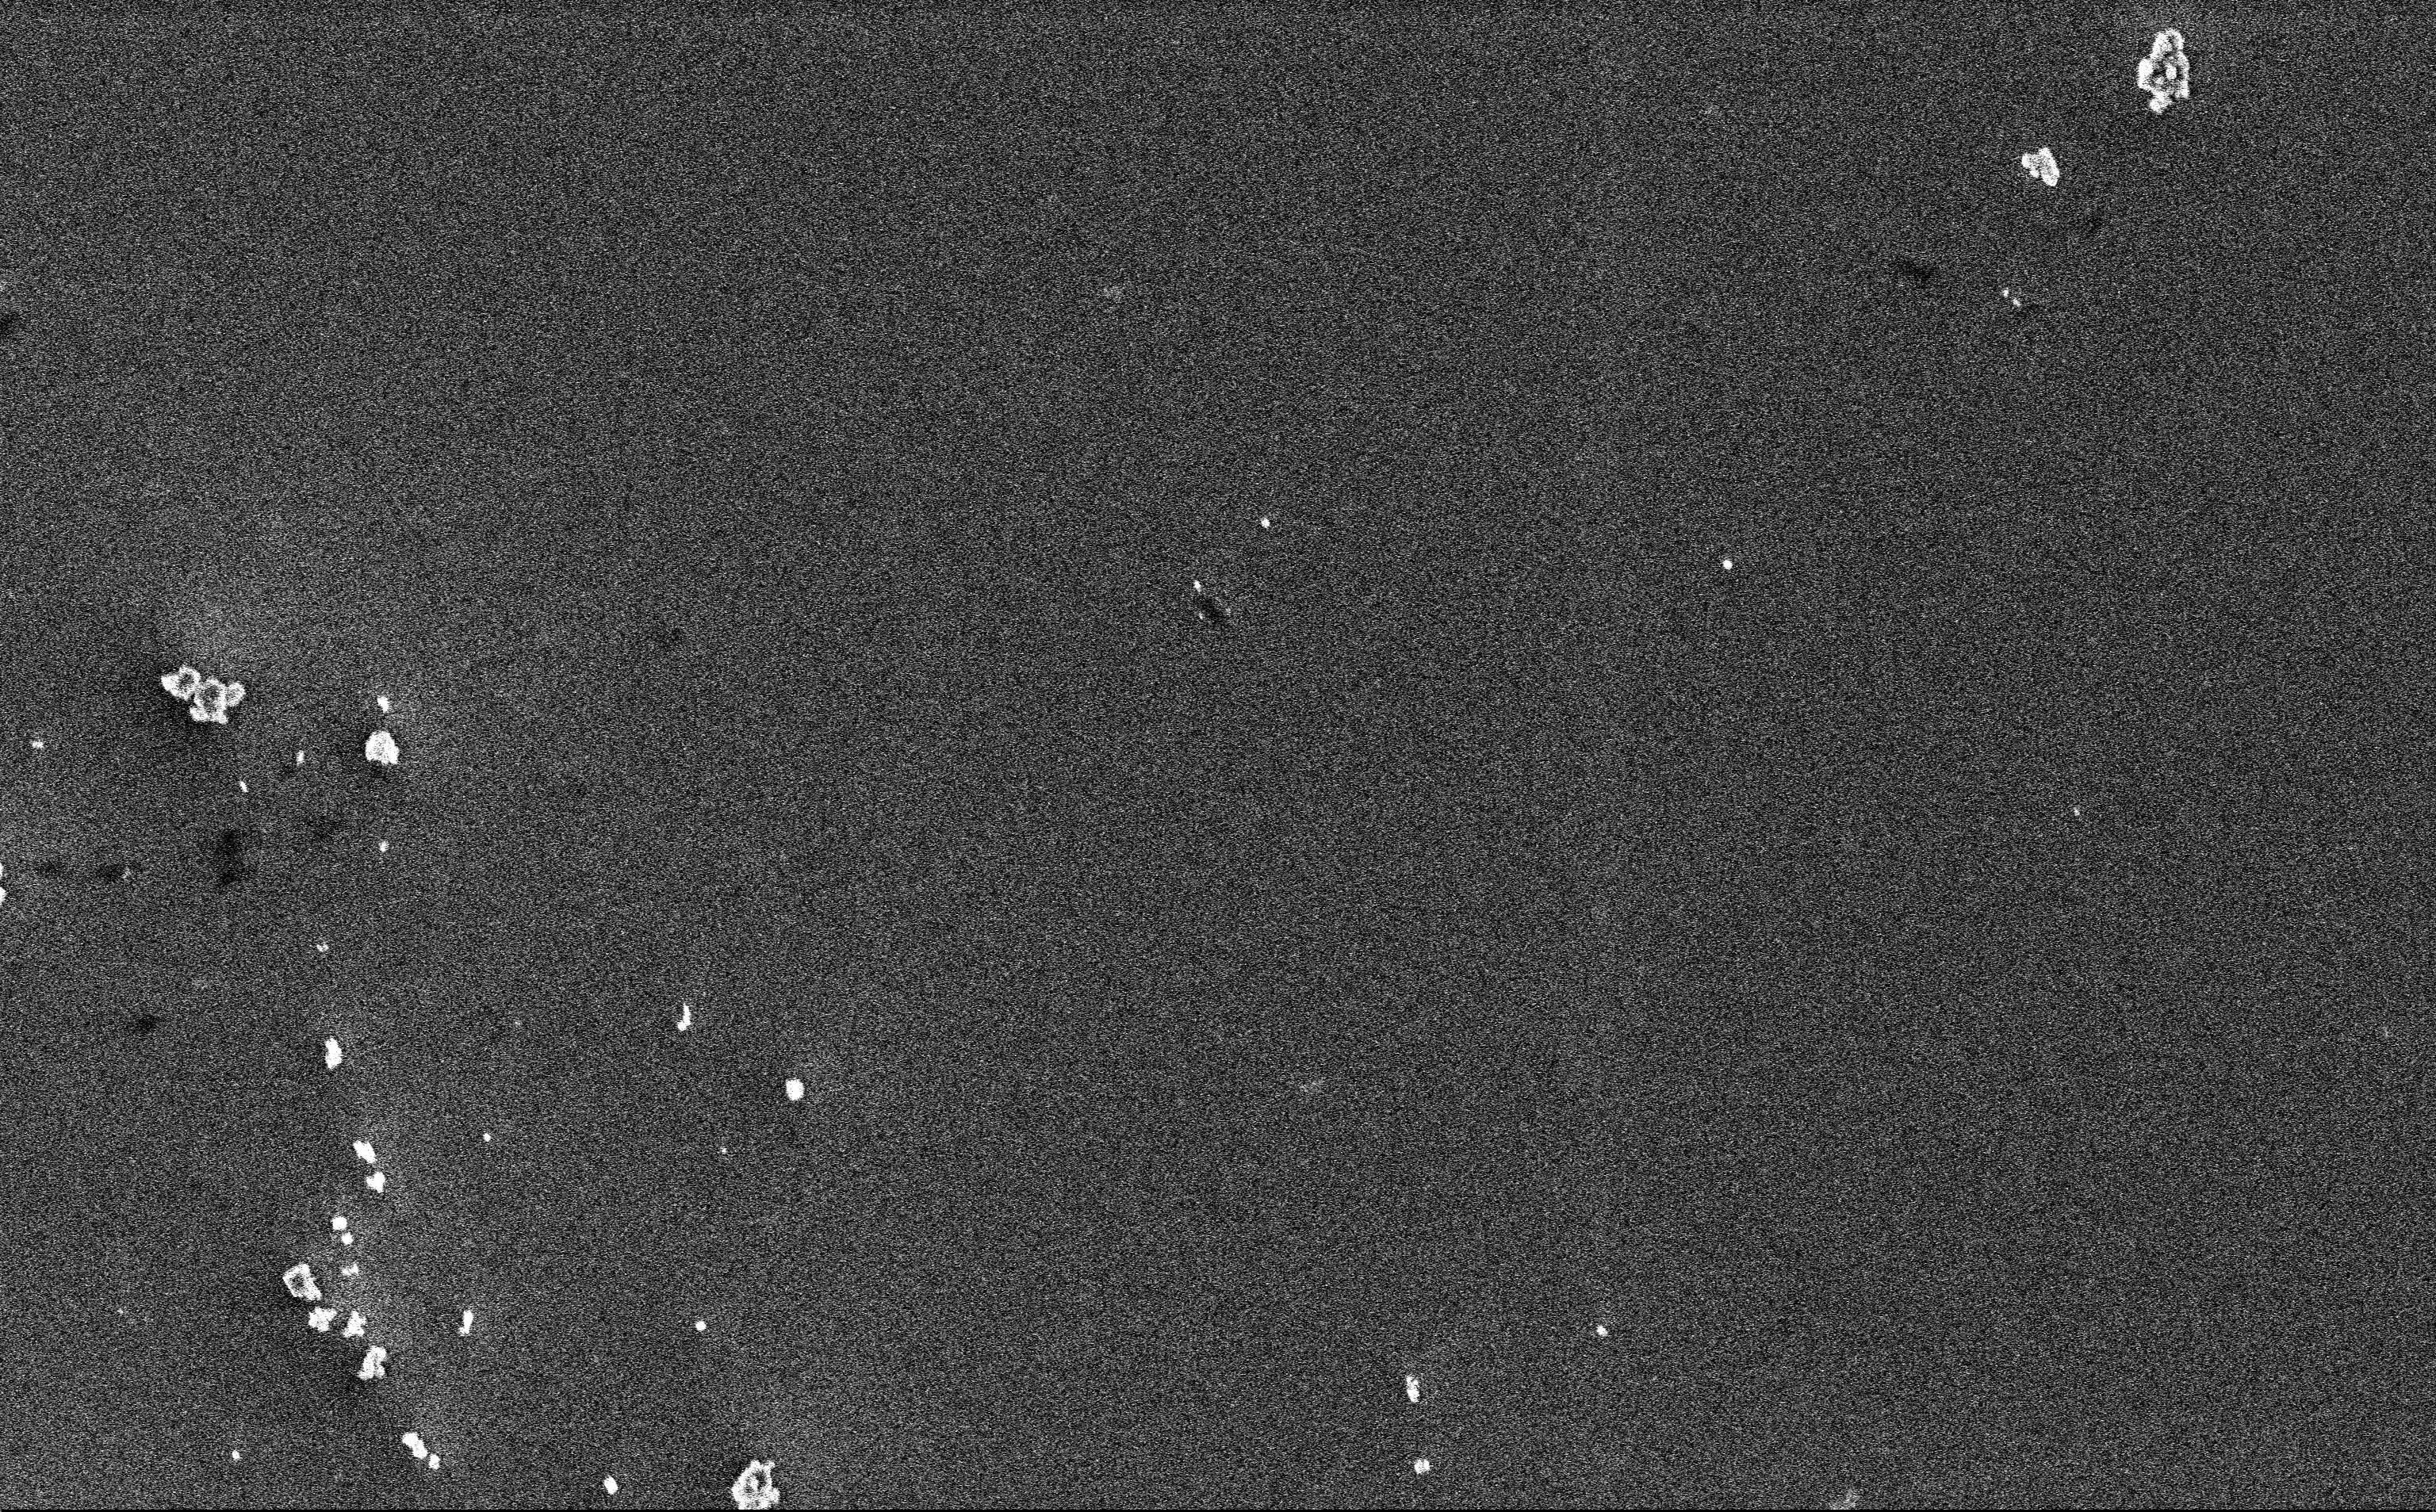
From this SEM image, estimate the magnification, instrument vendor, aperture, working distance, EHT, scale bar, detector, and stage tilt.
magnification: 12.85 K X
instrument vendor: Zeiss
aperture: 30 µm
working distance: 3 mm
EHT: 3 kV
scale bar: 2000 nm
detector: InLens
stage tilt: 0°